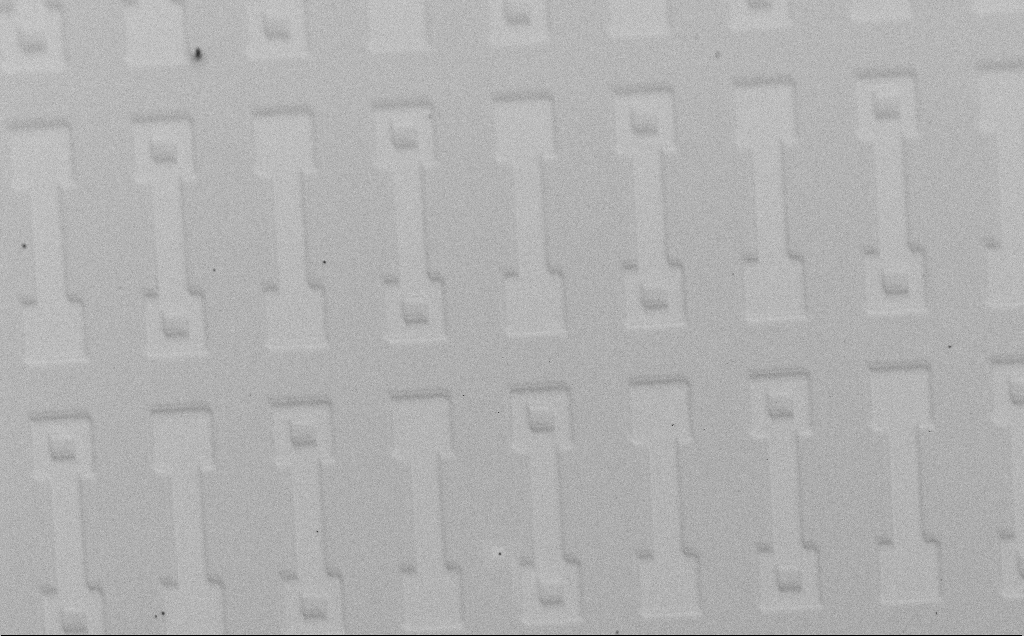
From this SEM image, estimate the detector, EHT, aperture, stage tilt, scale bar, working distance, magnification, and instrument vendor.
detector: SE2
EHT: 1.5 kV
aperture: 30 µm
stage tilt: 45°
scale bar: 100000 nm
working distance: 4 mm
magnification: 0.444 K X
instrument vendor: Zeiss